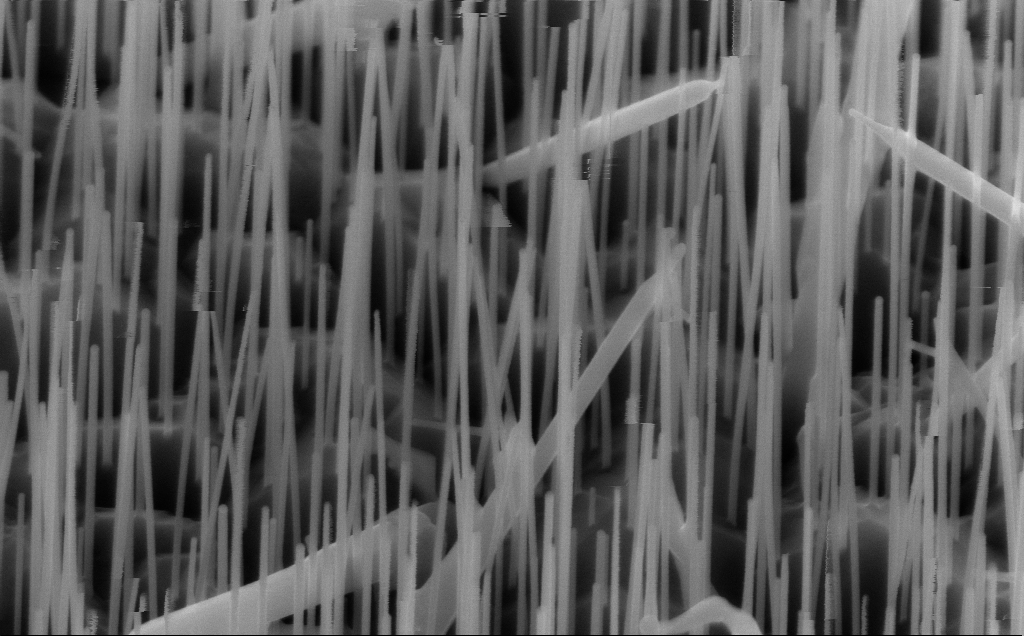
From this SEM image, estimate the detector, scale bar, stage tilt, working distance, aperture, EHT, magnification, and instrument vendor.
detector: InLens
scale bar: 200 nm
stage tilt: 45°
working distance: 6 mm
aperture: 30 µm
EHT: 10 kV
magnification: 80 K X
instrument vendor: Zeiss